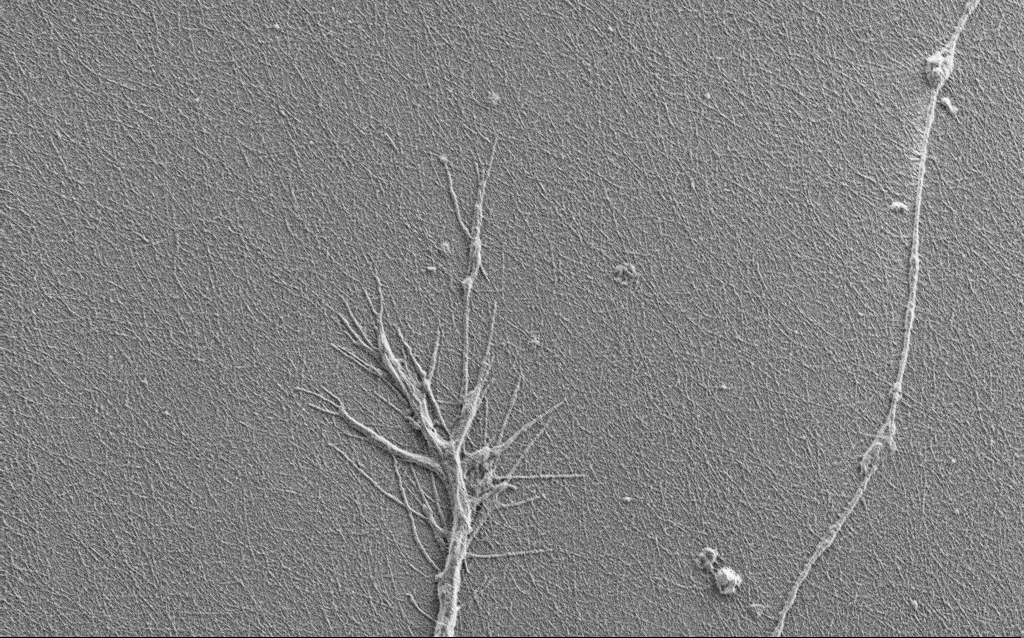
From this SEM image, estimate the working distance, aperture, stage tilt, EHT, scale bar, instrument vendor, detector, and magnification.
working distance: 4 mm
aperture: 30 µm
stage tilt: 0°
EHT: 1 kV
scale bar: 2000 nm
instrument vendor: Zeiss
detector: SE2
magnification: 7.5 K X